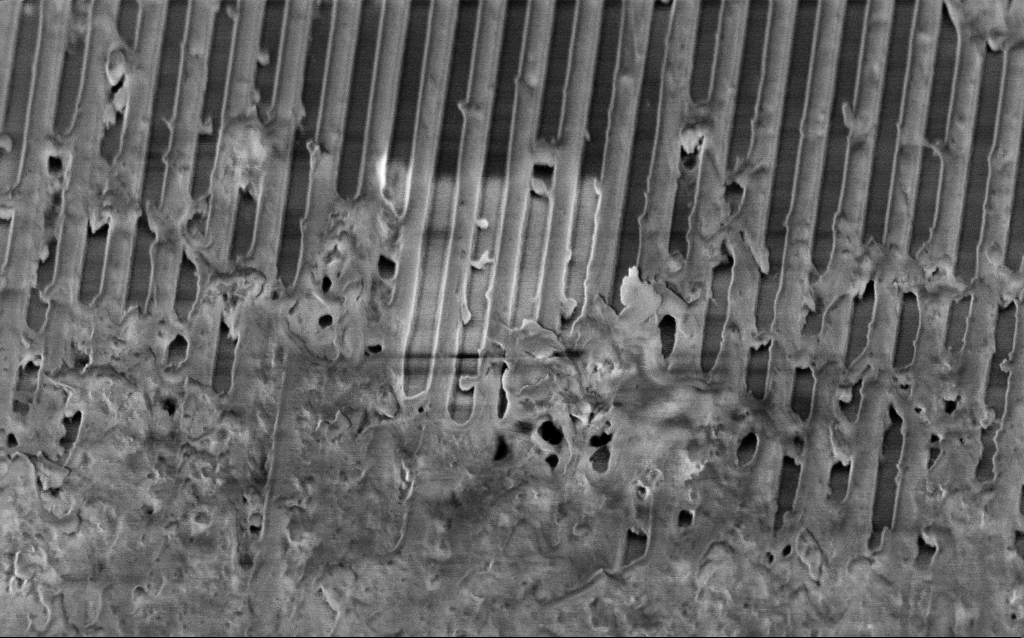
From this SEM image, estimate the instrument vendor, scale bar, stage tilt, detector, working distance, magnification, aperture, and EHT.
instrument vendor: Zeiss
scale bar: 1000 nm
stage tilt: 45°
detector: InLens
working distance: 4.1 mm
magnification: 35.53 K X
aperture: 30 µm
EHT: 2 kV